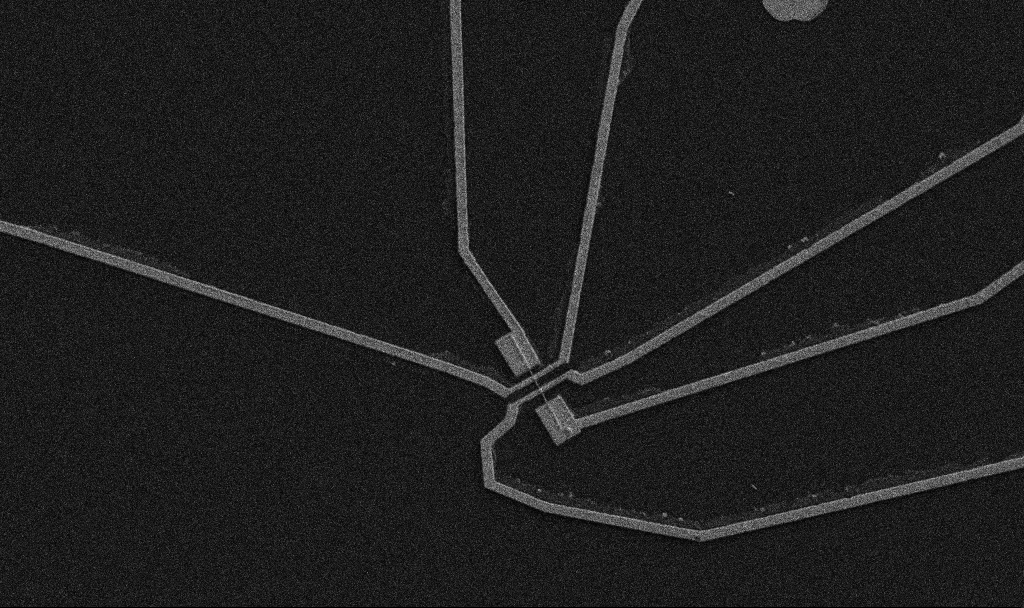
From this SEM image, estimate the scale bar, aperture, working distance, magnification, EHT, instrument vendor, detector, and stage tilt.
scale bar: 10000 nm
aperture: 30 µm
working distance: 10.7 mm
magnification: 5 K X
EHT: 5 kV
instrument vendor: Zeiss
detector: SE2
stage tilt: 0°